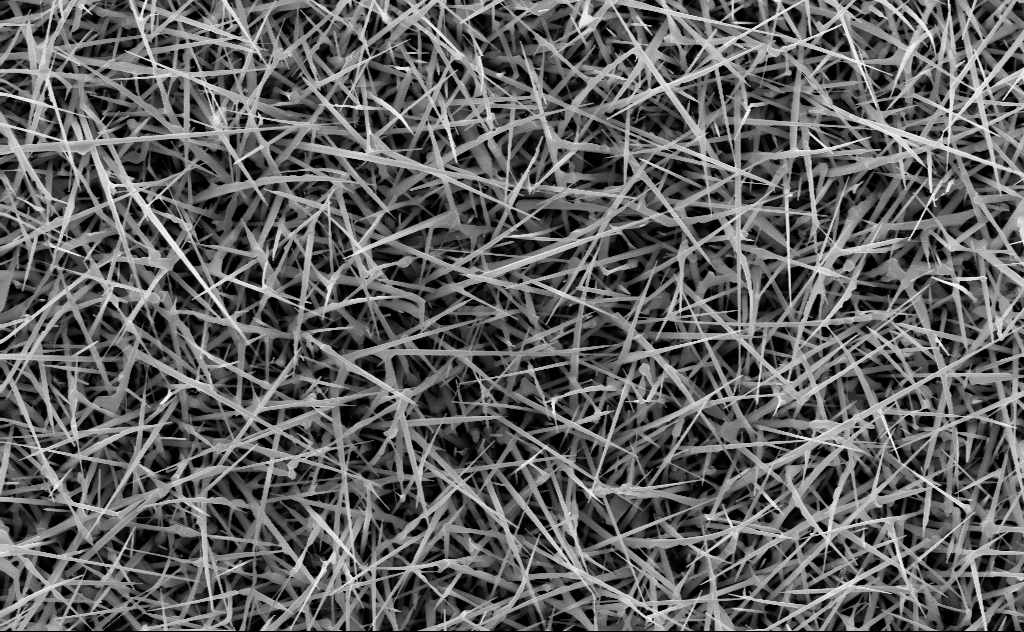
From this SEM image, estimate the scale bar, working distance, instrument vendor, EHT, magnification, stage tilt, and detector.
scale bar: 1000 nm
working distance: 10 mm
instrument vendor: Zeiss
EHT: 10 kV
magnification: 20 K X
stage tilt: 0°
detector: InLens